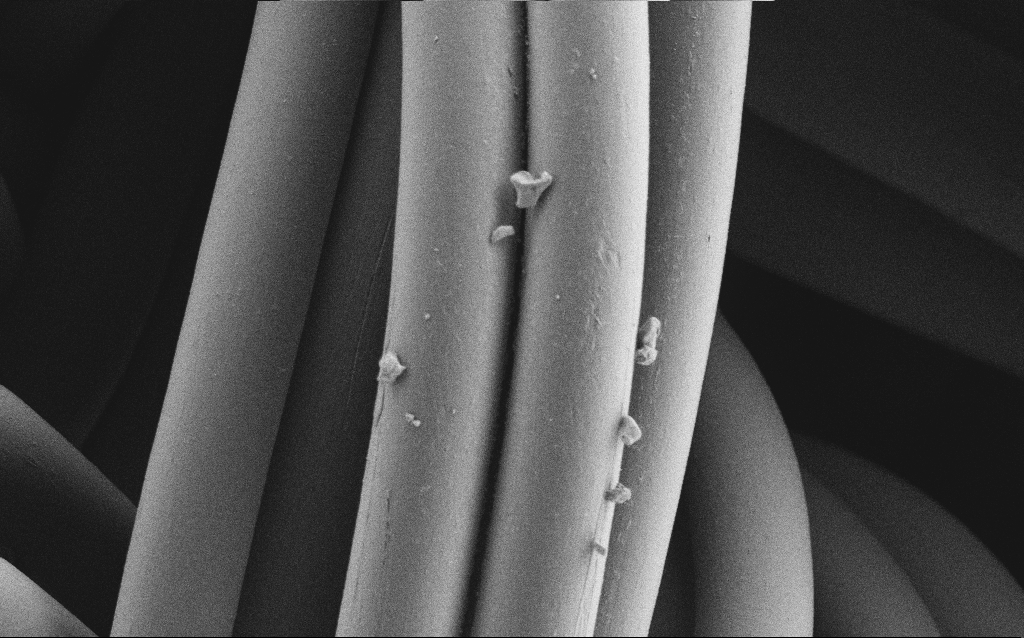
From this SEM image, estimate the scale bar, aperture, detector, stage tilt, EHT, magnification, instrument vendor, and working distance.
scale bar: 20000 nm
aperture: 30 µm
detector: SE2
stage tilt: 0°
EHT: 1 kV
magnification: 1.87 K X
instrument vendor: Zeiss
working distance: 4 mm